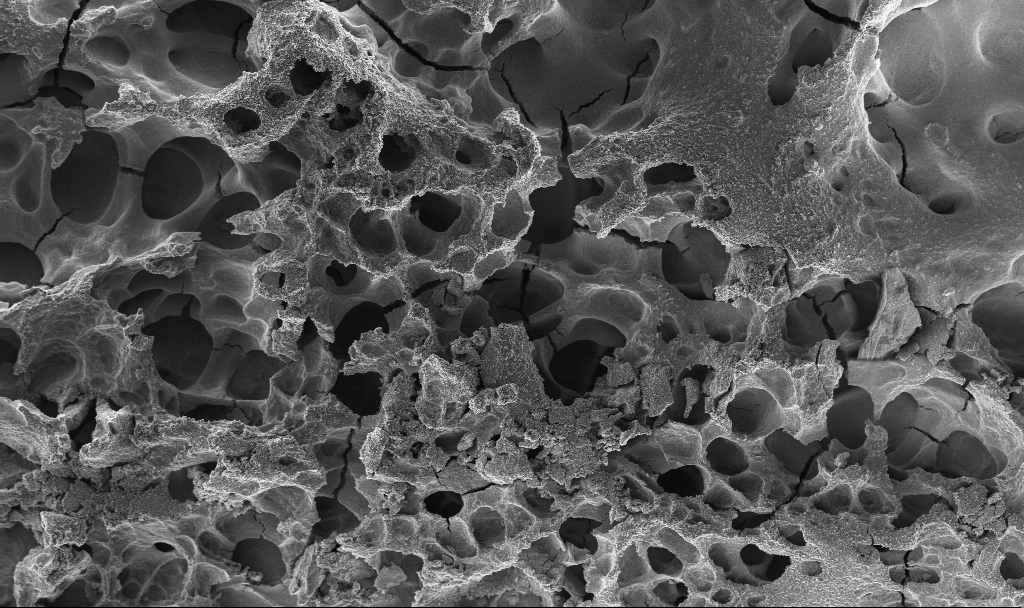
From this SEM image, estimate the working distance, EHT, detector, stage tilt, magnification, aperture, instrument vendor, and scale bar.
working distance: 2.4 mm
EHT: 3 kV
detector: InLens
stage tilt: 0°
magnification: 1 K X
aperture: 30 µm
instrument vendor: Zeiss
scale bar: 20000 nm